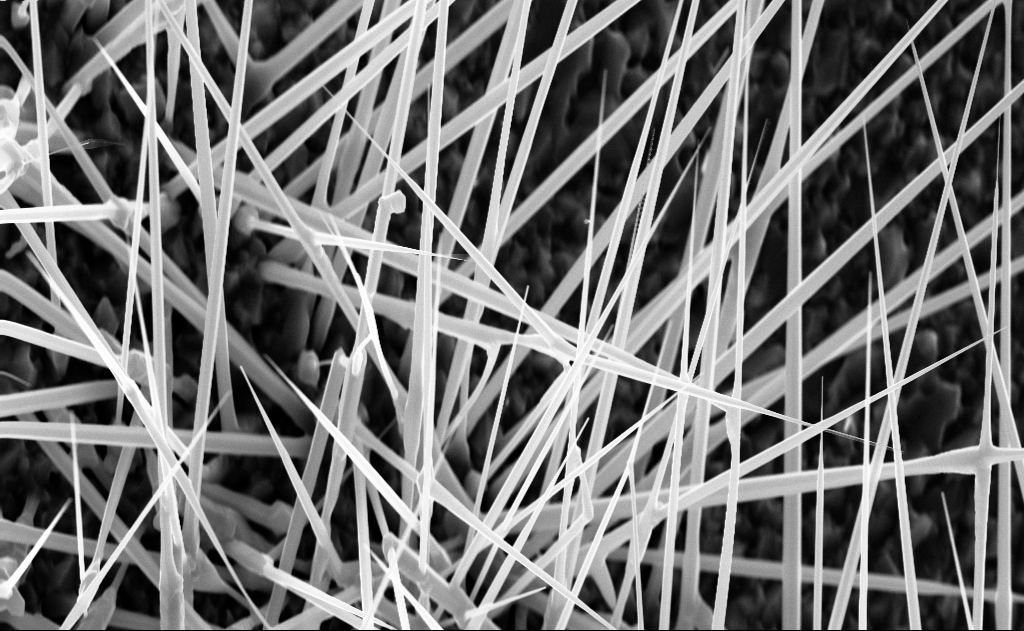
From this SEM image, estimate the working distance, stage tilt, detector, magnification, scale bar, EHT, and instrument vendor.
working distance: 4 mm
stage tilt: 0°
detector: InLens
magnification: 20 K X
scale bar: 2000 nm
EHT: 10 kV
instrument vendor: Zeiss